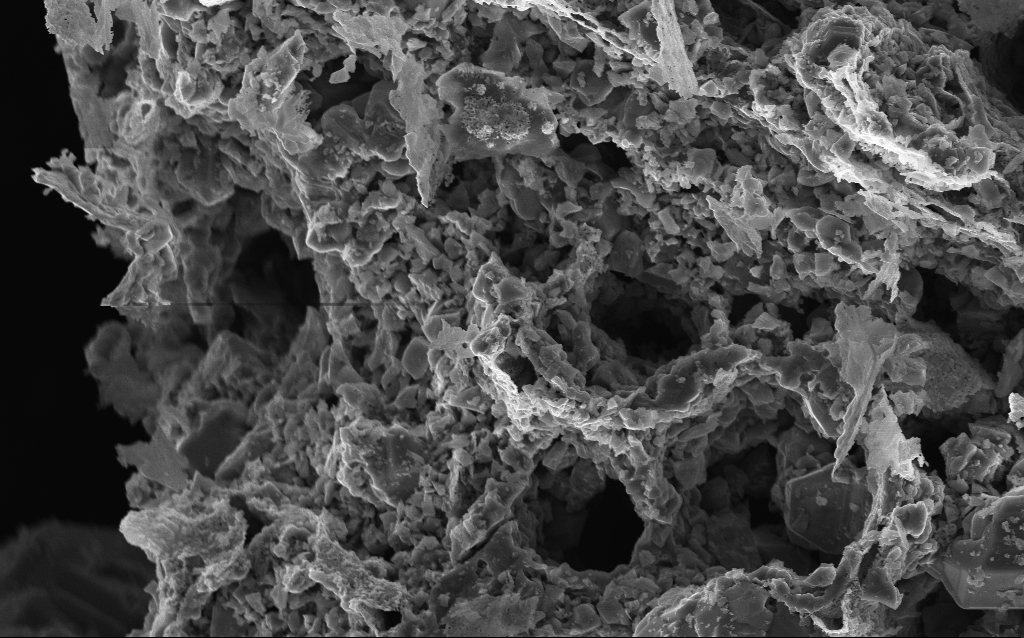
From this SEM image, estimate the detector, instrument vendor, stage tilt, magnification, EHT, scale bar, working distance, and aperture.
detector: InLens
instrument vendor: Zeiss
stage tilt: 0°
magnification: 5 K X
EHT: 10 kV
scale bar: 10000 nm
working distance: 3 mm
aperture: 30 µm